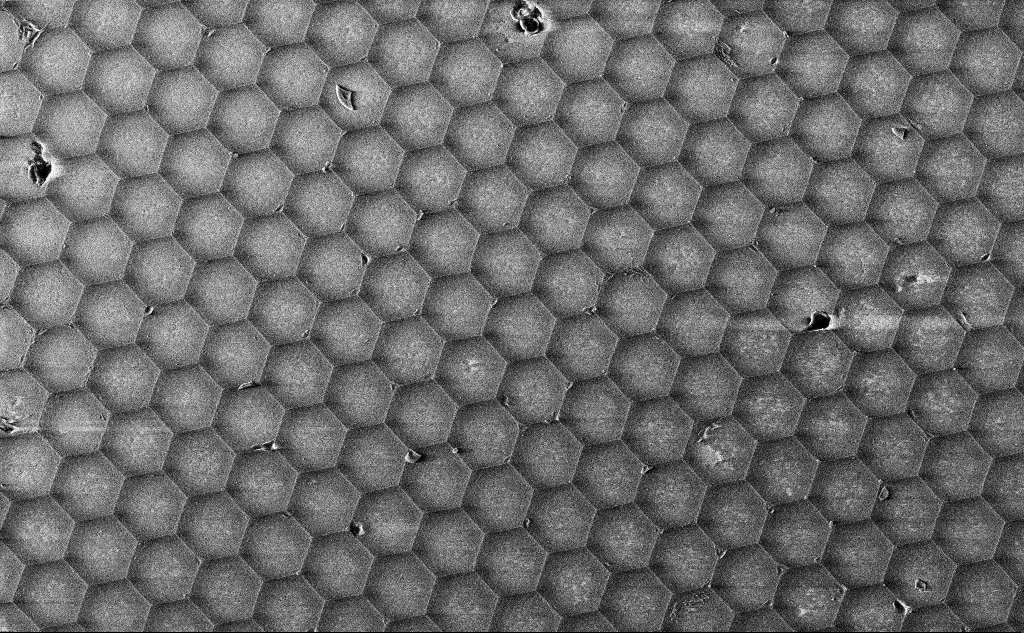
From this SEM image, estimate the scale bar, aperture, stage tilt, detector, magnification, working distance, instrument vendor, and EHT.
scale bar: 2000 nm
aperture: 30 µm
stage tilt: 0°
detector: InLens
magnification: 7.97 K X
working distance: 6.6 mm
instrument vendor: Zeiss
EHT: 3 kV